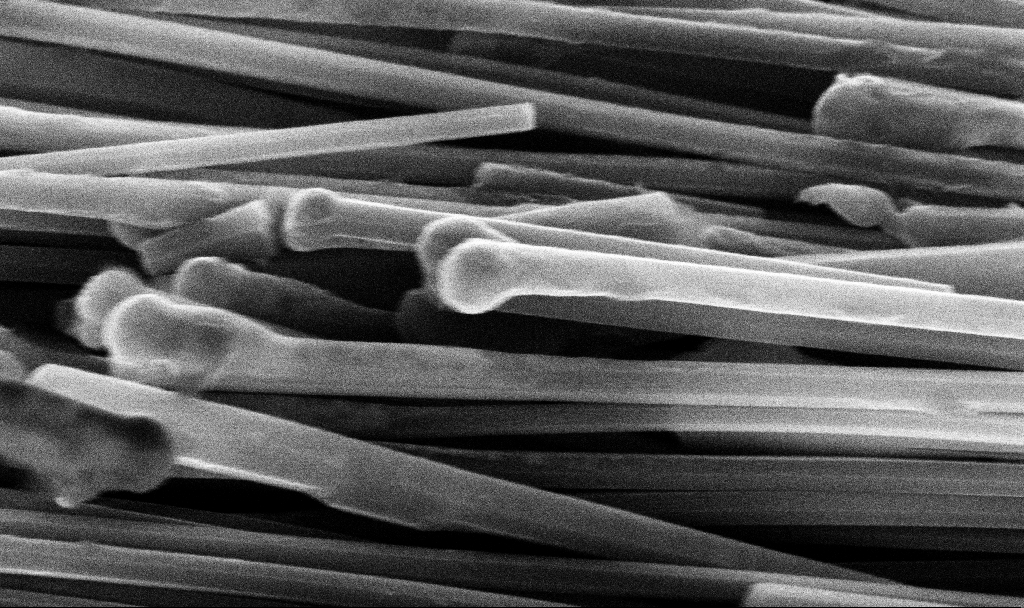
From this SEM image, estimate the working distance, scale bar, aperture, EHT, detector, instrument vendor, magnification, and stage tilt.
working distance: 7.2 mm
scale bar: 200 nm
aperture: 30 µm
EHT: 10 kV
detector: InLens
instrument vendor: Zeiss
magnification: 126.67 K X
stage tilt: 45°